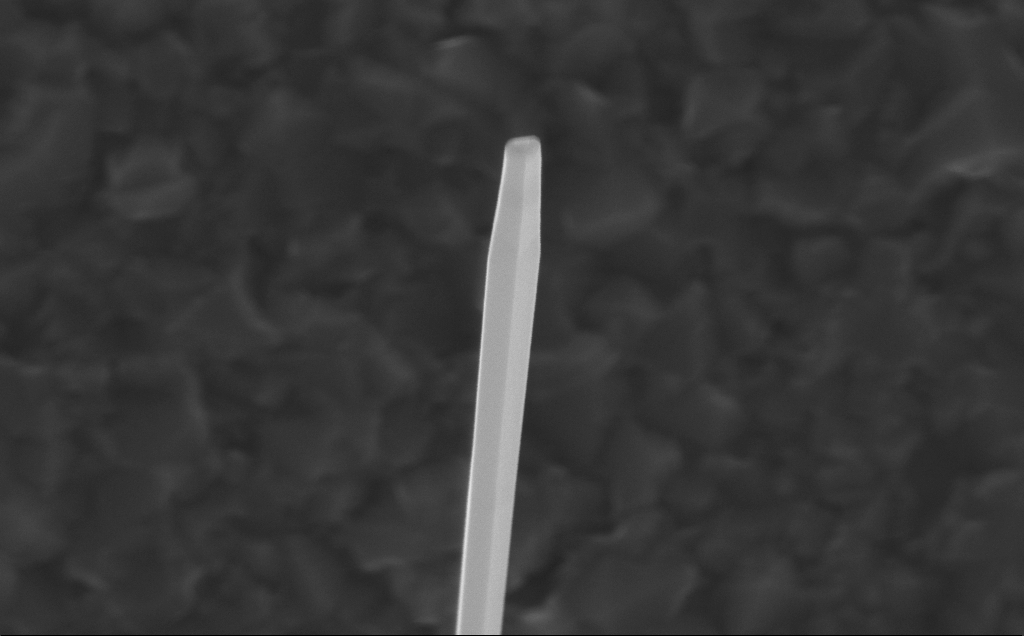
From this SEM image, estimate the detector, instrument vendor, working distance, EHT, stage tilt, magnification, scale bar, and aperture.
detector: InLens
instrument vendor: Zeiss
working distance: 5 mm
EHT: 10 kV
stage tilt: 0°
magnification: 80 K X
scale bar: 200 nm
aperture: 30 µm